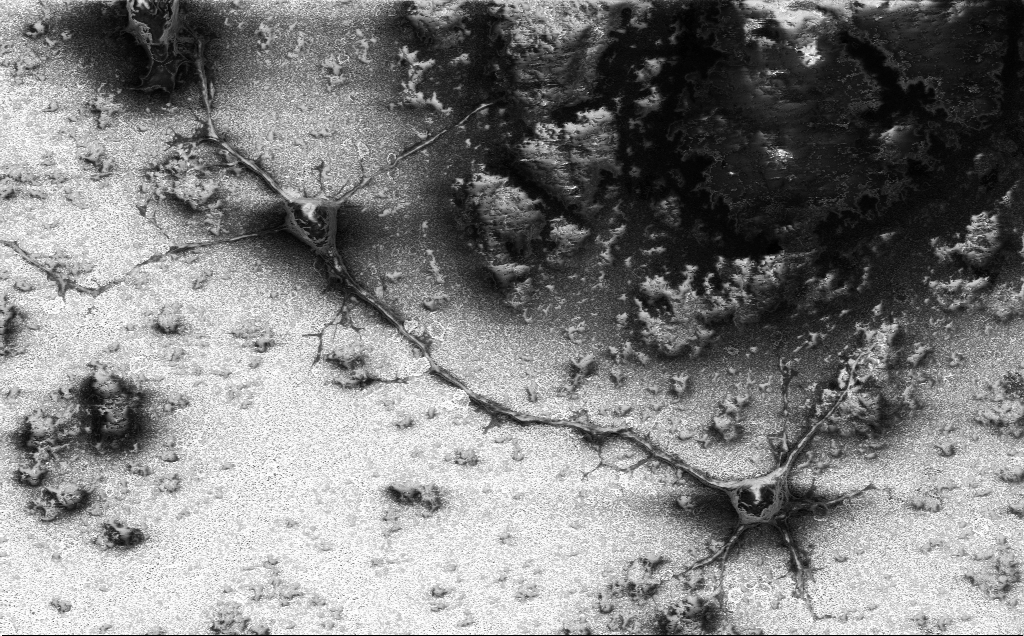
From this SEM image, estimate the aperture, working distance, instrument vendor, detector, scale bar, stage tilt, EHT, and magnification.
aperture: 30 µm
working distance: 7.1 mm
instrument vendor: Zeiss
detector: InLens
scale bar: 10000 nm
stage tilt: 0°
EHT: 2 kV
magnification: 3 K X